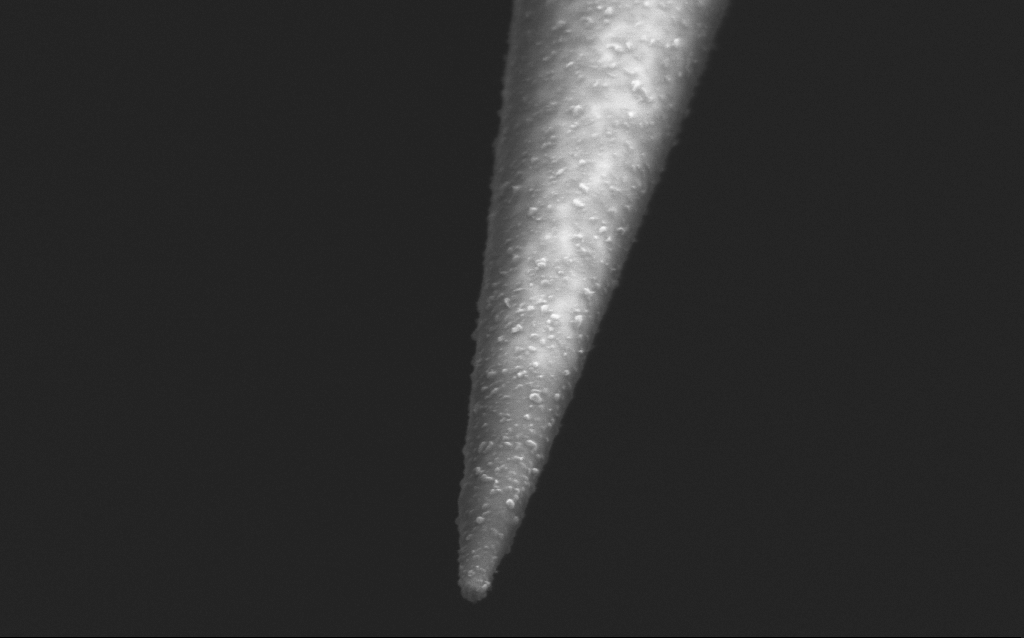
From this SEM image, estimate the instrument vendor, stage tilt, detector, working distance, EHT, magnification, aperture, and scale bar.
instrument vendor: Zeiss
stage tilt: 45°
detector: InLens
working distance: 5 mm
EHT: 2.5 kV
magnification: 100 K X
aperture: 30 µm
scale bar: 200 nm